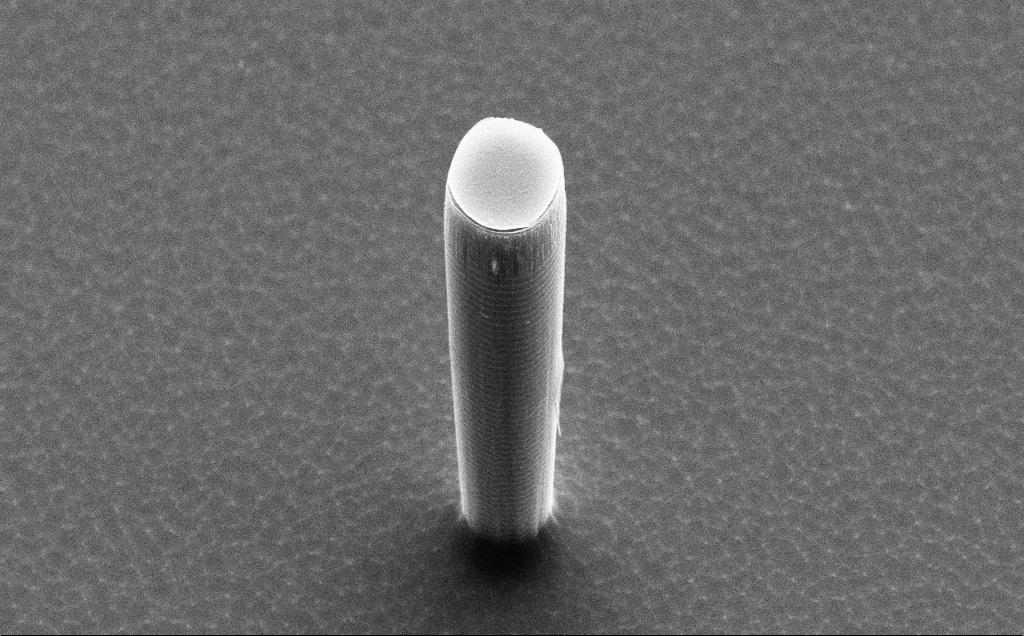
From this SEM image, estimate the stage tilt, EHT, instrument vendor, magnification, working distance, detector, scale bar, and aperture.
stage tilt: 50°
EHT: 15 kV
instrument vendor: Zeiss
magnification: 6.45 K X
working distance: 10 mm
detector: SE2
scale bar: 10000 nm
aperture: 30 µm